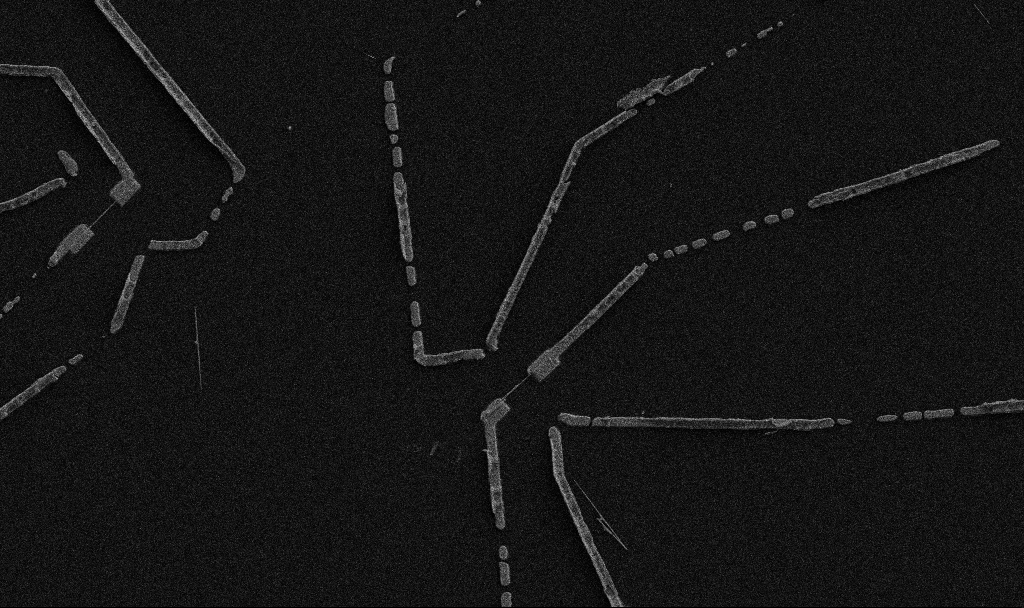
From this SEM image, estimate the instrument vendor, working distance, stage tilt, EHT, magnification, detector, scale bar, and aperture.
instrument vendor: Zeiss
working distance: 10.7 mm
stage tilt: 0°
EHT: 5 kV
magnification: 5 K X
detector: SE2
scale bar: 10000 nm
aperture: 30 µm